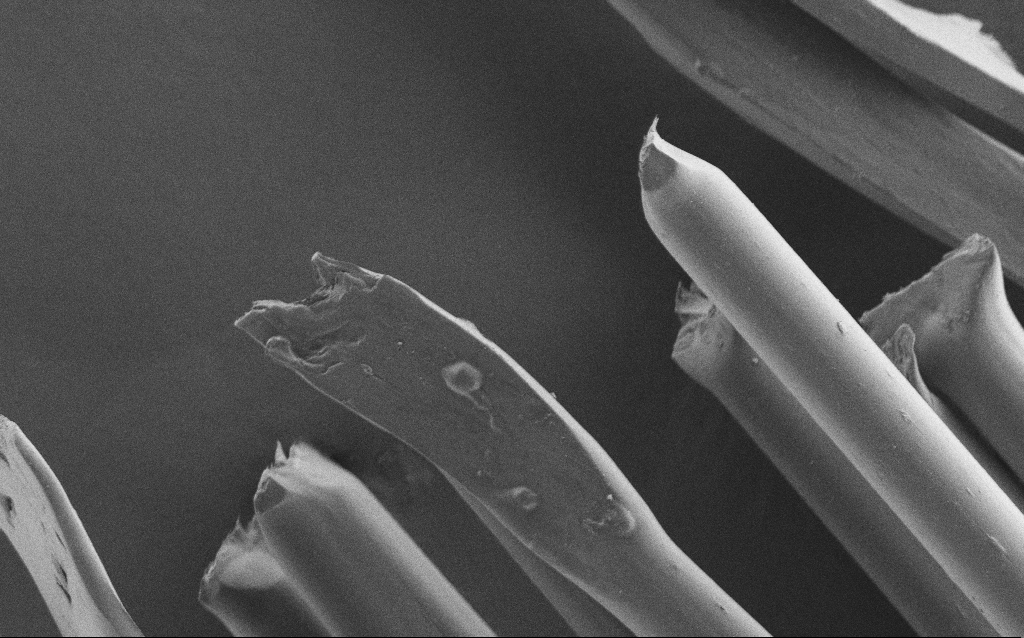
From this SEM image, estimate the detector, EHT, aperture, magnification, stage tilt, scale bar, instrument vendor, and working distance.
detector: SE2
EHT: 1 kV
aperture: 30 µm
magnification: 2.43 K X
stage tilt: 0°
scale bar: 10000 nm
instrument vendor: Zeiss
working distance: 5 mm